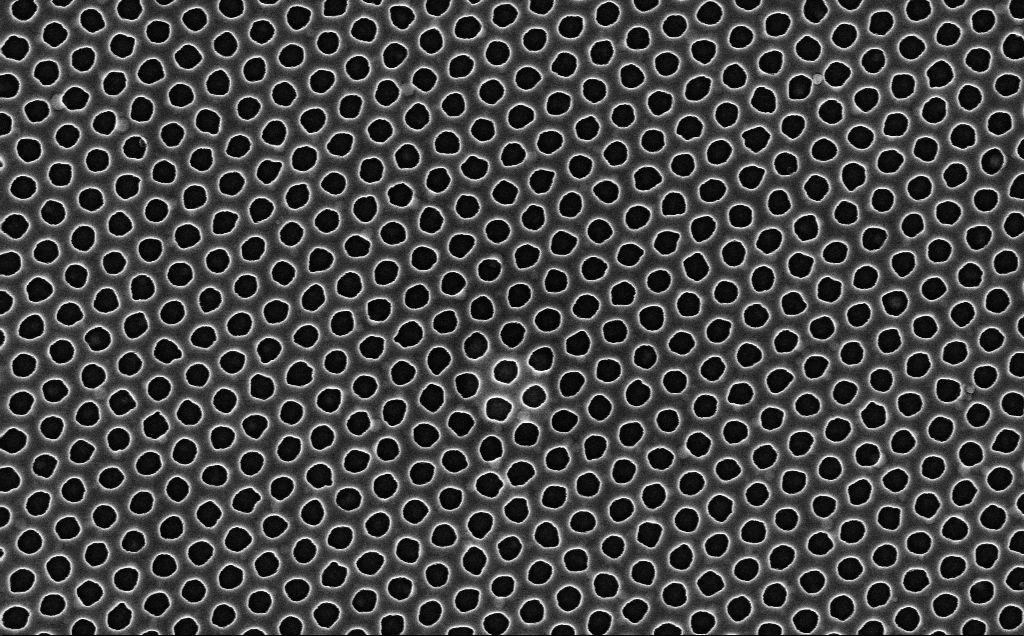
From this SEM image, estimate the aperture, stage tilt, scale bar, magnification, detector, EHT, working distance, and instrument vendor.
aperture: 30 µm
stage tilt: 0°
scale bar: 1000 nm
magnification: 40 K X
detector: InLens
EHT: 3 kV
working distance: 2.5 mm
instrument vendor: Zeiss